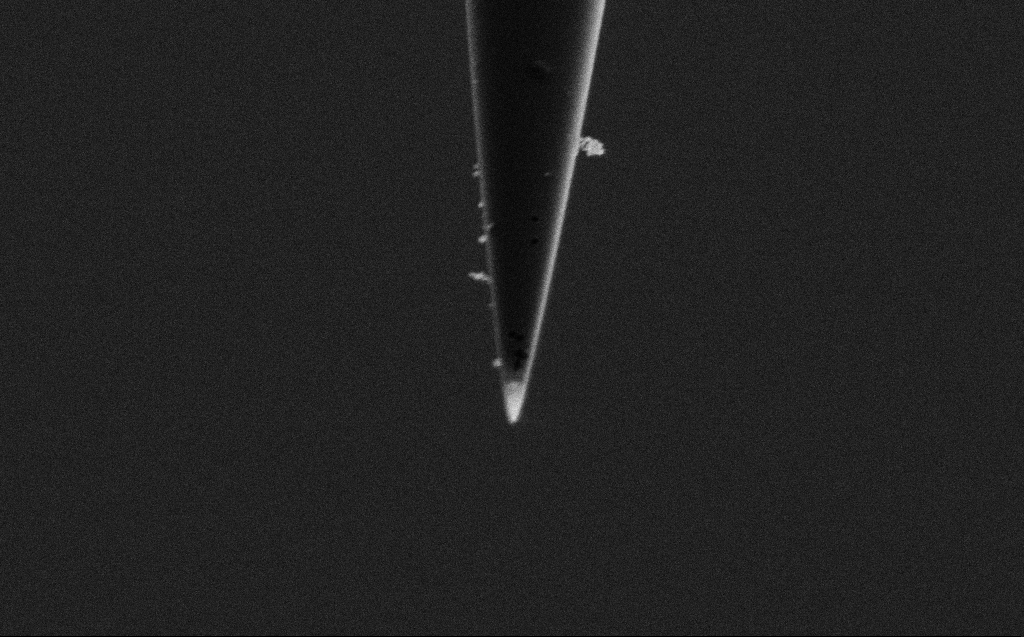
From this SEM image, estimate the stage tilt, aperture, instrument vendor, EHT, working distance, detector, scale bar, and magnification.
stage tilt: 45.1°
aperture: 20 µm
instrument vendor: Zeiss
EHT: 2 kV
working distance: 4 mm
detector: SE2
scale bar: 1000 nm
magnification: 29.77 K X